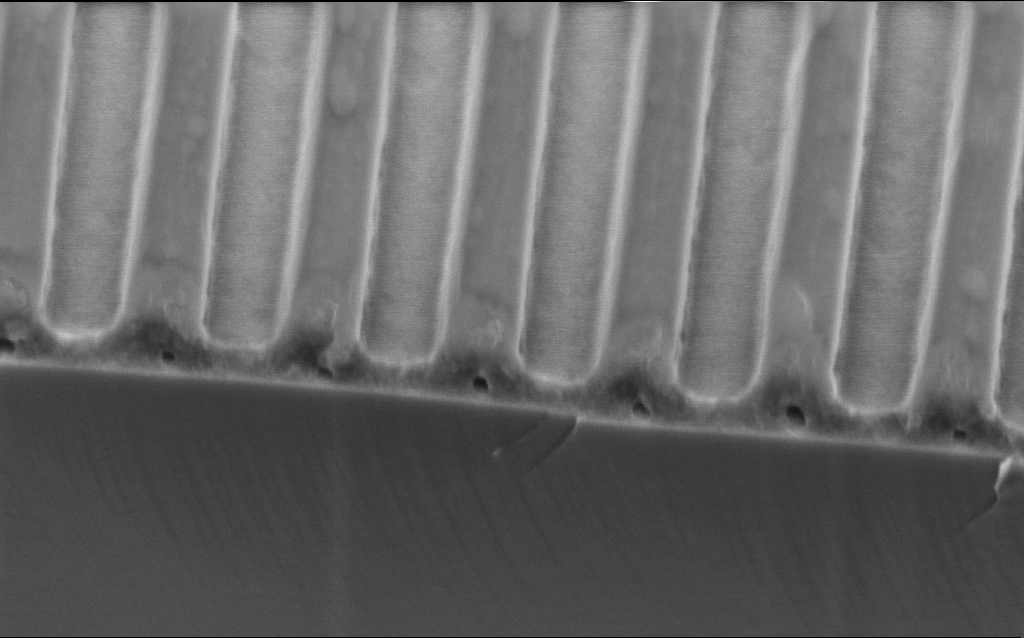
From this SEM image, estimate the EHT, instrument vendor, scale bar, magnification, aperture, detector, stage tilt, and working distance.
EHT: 2 kV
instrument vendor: Zeiss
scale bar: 200 nm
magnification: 117.05 K X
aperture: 30 µm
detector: InLens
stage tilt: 45°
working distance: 3.8 mm